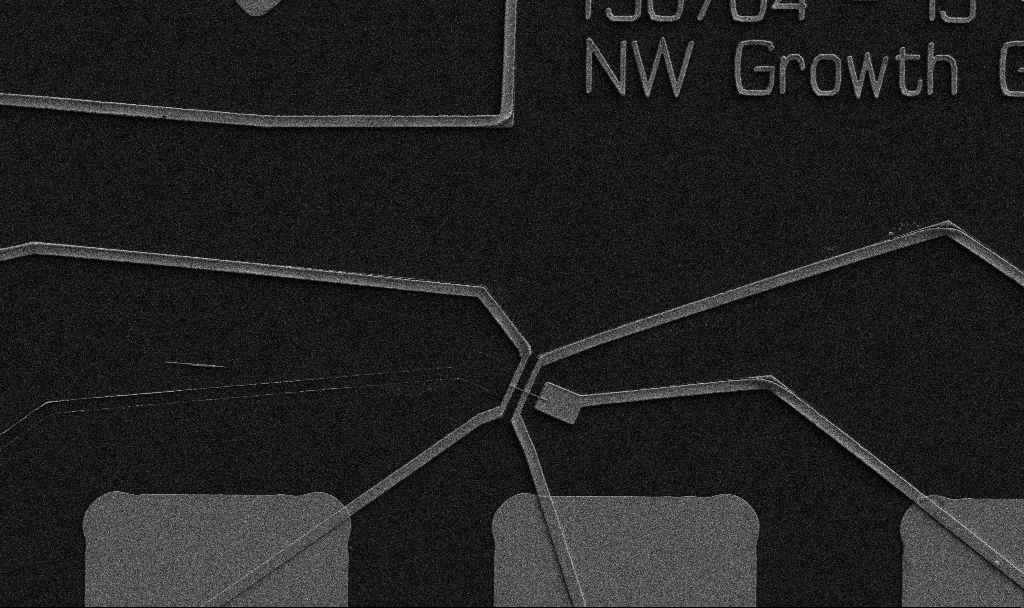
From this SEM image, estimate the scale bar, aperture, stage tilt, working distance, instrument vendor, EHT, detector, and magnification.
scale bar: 10000 nm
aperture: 30 µm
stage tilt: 0°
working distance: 10.7 mm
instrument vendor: Zeiss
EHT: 5 kV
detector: SE2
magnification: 5 K X